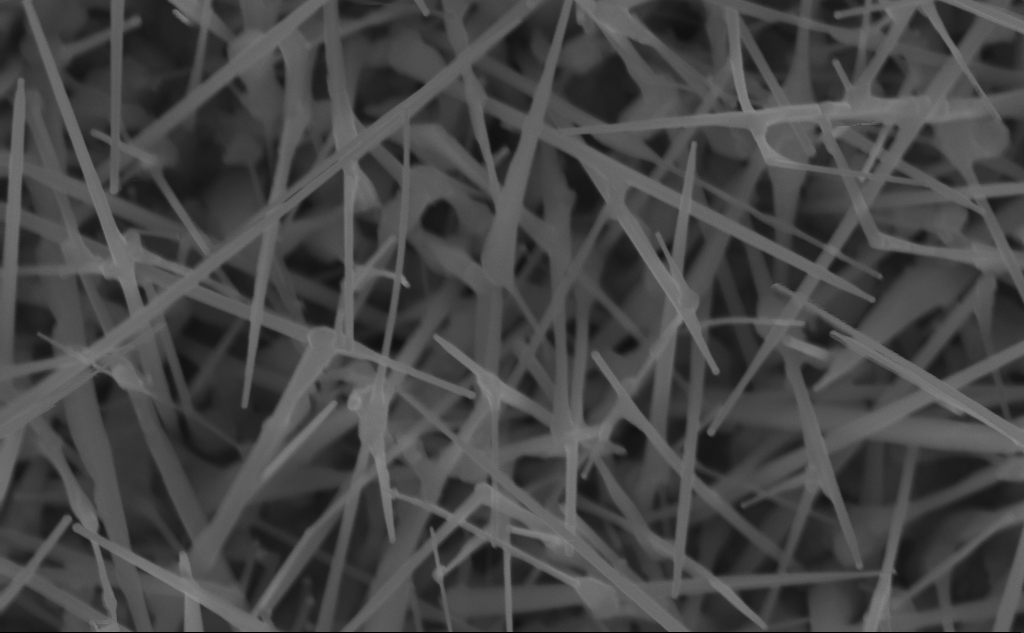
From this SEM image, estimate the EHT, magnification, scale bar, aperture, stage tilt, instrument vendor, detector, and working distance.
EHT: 10 kV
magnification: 80 K X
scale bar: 200 nm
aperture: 30 µm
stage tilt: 0°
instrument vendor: Zeiss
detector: InLens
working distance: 5 mm